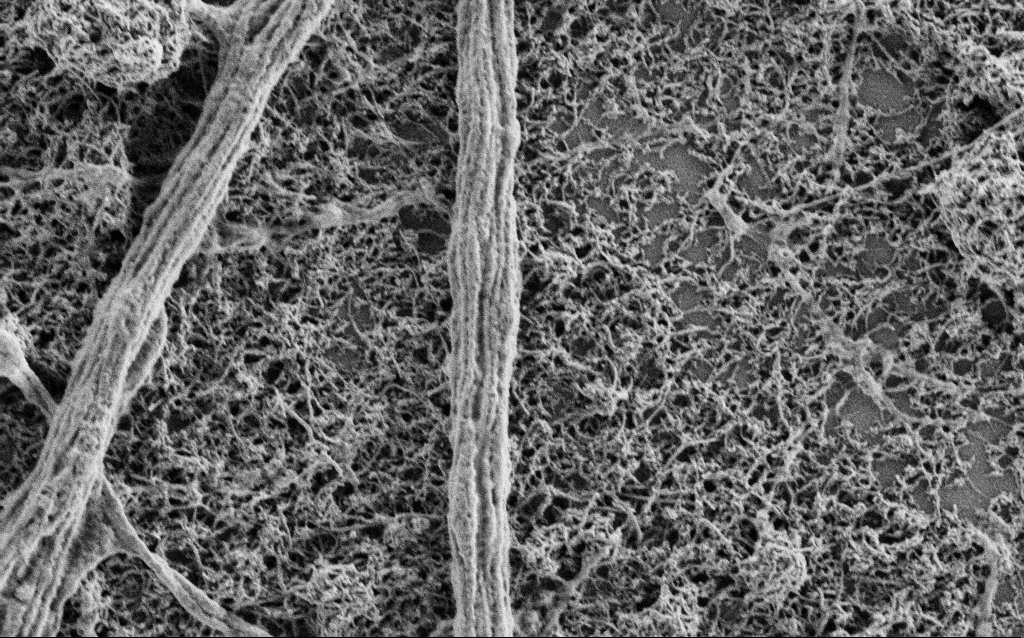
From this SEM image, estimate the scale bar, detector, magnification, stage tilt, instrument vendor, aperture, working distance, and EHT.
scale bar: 2000 nm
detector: SE2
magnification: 25 K X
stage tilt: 0°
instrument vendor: Zeiss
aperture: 30 µm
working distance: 4 mm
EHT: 1 kV